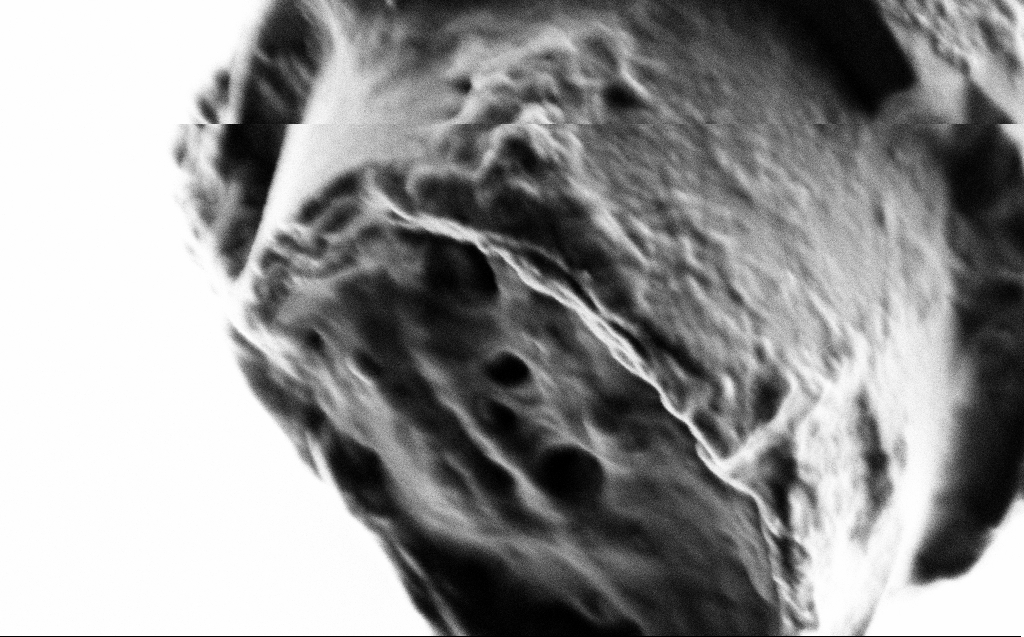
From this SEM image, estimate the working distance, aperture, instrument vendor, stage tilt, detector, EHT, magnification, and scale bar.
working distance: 2 mm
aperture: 30 µm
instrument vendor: Zeiss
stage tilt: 45°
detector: SE2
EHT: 1 kV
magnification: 102.8 K X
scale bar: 200 nm